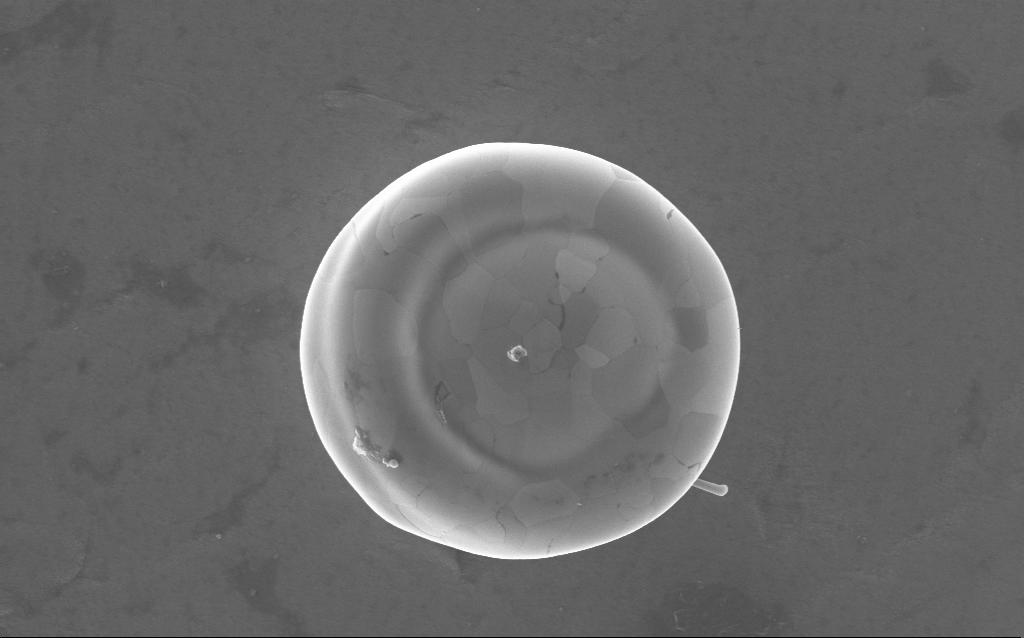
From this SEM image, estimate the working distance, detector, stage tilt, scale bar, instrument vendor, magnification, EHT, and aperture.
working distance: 3 mm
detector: InLens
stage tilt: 0°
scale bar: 2000 nm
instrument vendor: Zeiss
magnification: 36 K X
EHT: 5 kV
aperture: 30 µm